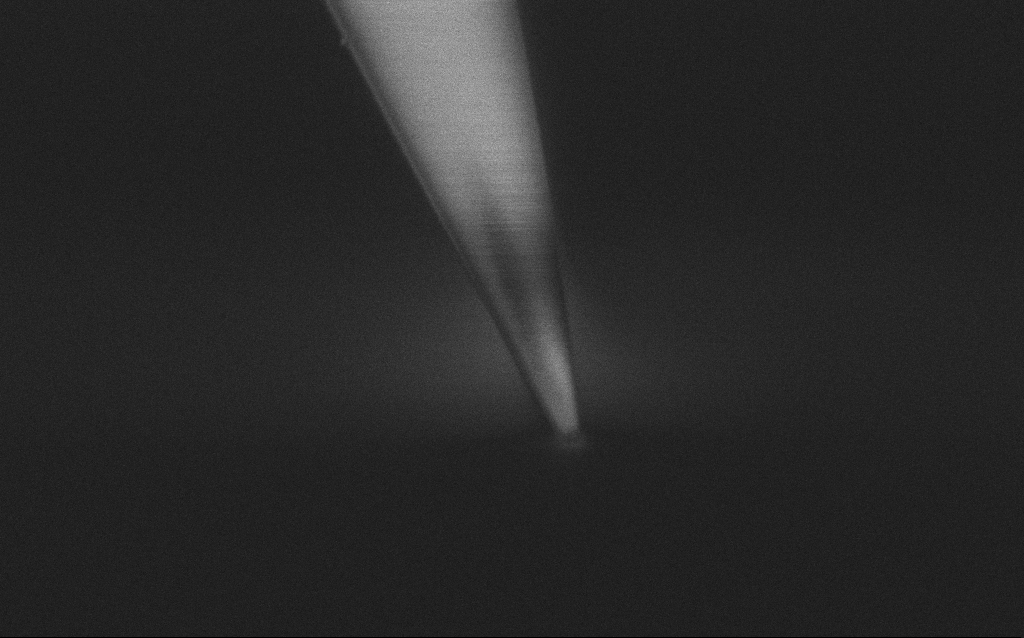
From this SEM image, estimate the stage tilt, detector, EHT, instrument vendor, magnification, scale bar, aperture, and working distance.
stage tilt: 45°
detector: InLens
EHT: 1 kV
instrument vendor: Zeiss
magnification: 50 K X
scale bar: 1000 nm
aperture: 30 µm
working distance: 7 mm